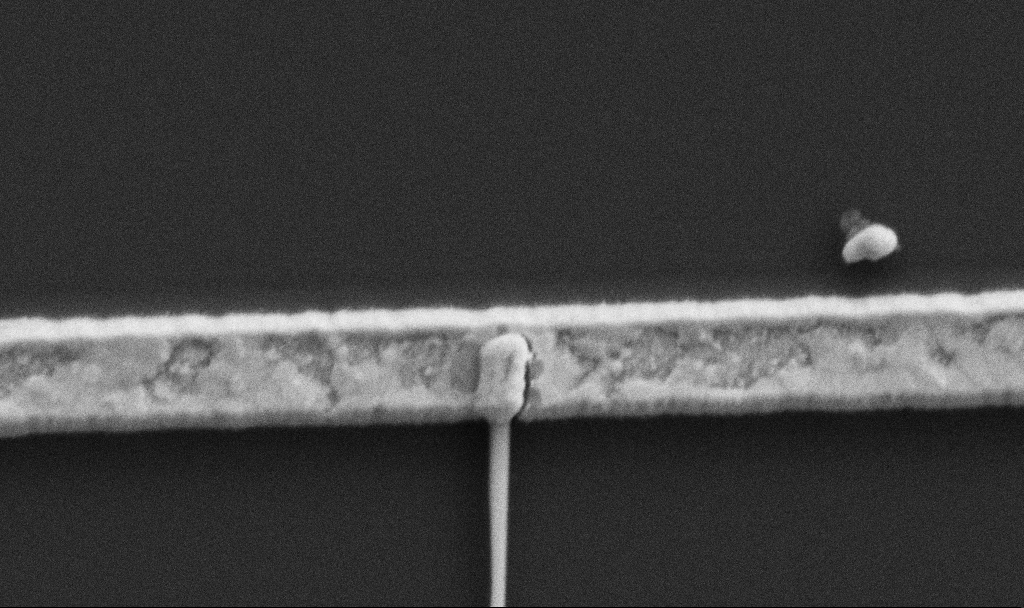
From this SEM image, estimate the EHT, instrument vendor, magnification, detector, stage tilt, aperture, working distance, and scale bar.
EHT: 5 kV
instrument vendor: Zeiss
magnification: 60 K X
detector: SE2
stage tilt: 0°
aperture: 30 µm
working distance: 10.7 mm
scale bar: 1000 nm